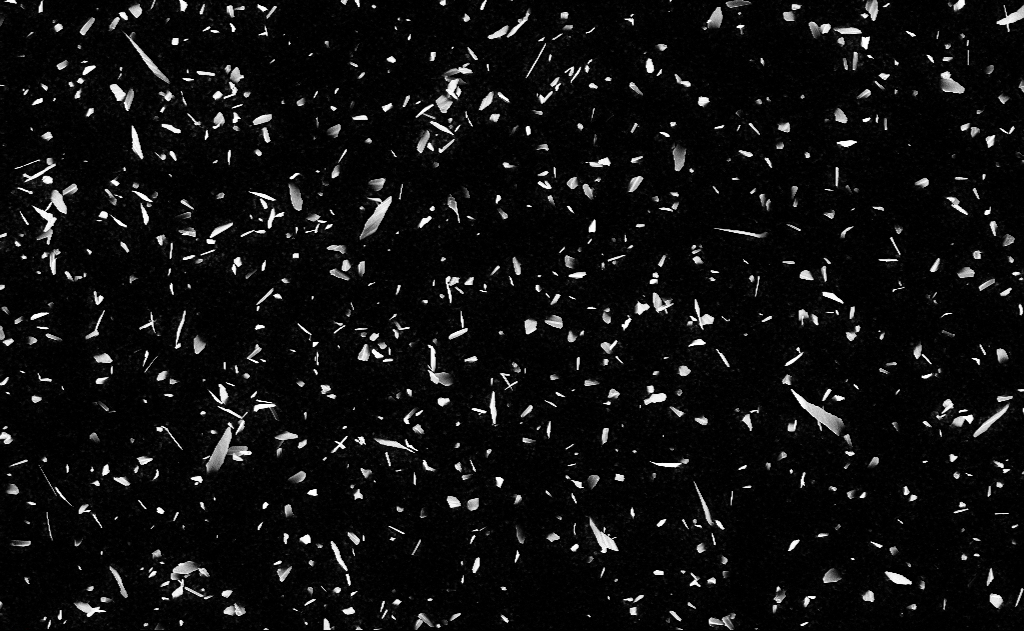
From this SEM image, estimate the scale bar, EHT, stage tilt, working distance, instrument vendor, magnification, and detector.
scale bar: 10000 nm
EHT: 10 kV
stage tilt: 0°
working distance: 13 mm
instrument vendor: Zeiss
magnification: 5 K X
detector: InLens